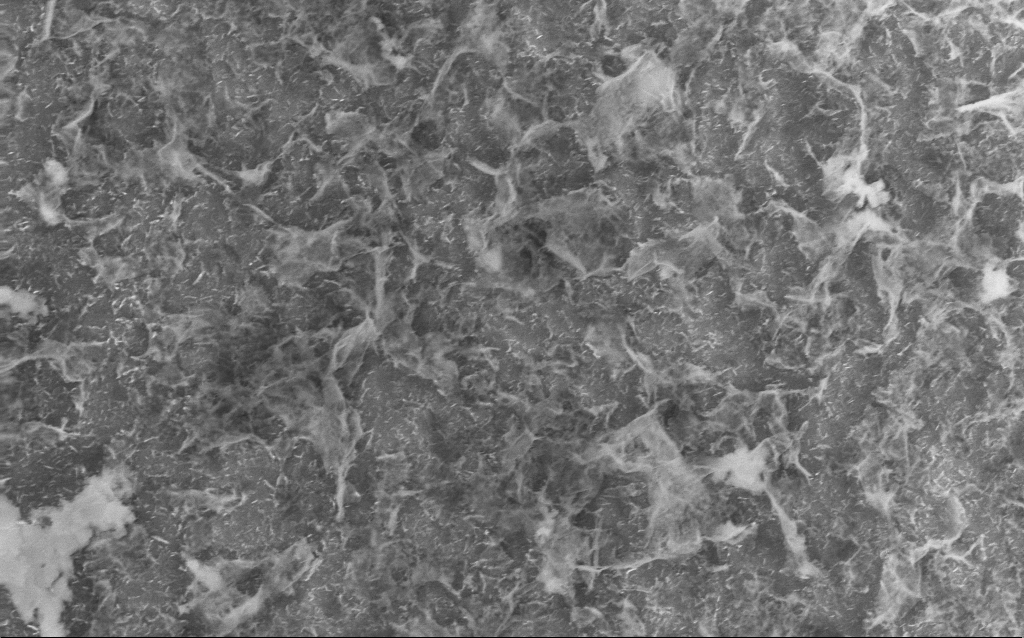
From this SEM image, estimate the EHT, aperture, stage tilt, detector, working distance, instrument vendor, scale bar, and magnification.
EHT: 10 kV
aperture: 30 µm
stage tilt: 0°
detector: InLens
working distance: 2.5 mm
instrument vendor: Zeiss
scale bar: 2000 nm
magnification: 20 K X